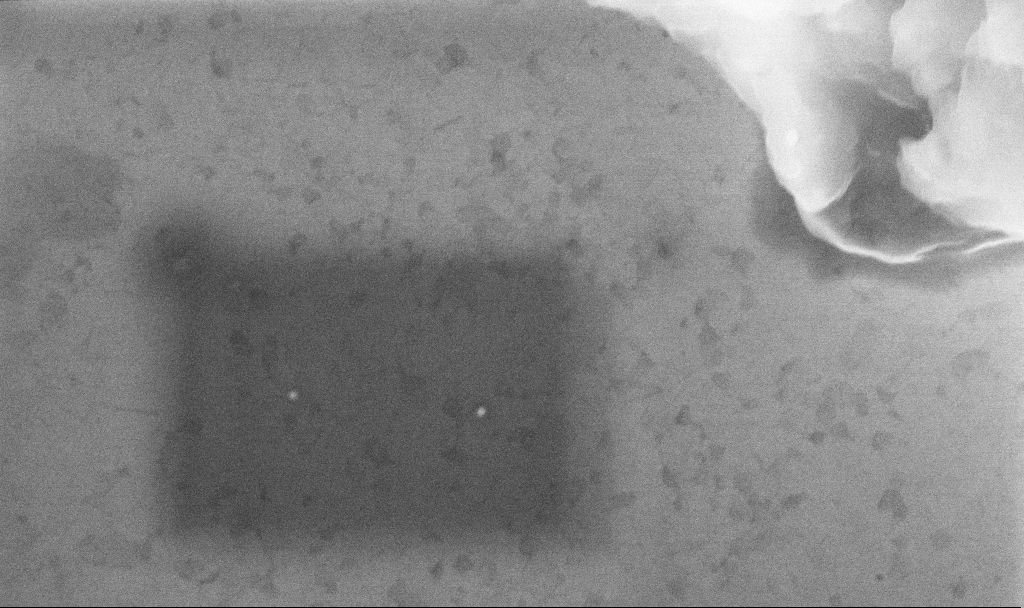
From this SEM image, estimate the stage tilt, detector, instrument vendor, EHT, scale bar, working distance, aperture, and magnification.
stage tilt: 0°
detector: InLens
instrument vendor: Zeiss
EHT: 10 kV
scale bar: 200 nm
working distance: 3.3 mm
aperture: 30 µm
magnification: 108.38 K X